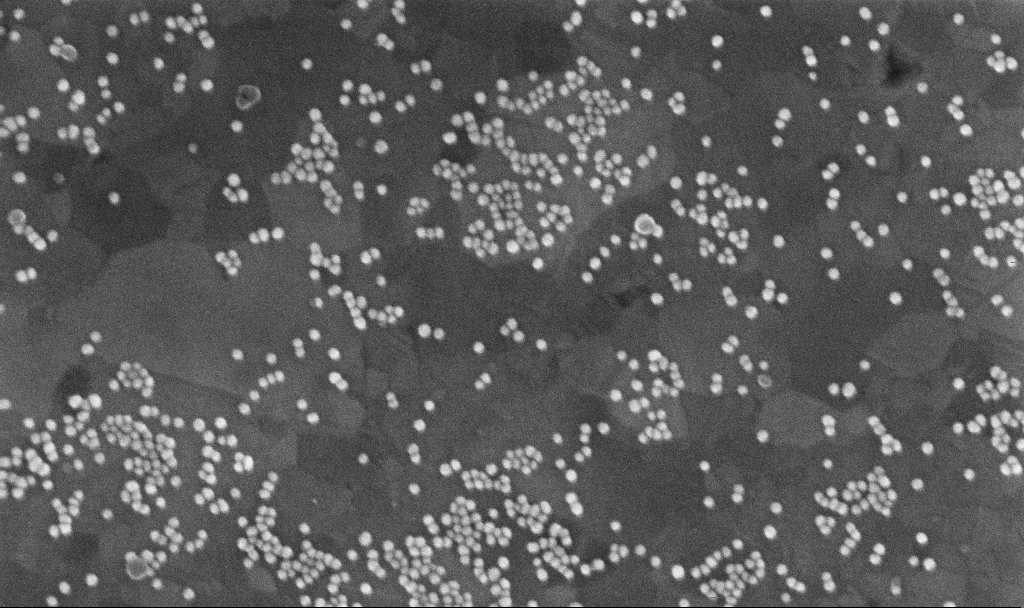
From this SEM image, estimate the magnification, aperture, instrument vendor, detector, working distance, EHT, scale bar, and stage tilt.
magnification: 200 K X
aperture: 30 µm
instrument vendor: Zeiss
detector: InLens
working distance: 3.7 mm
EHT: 10 kV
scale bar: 200 nm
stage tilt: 0°